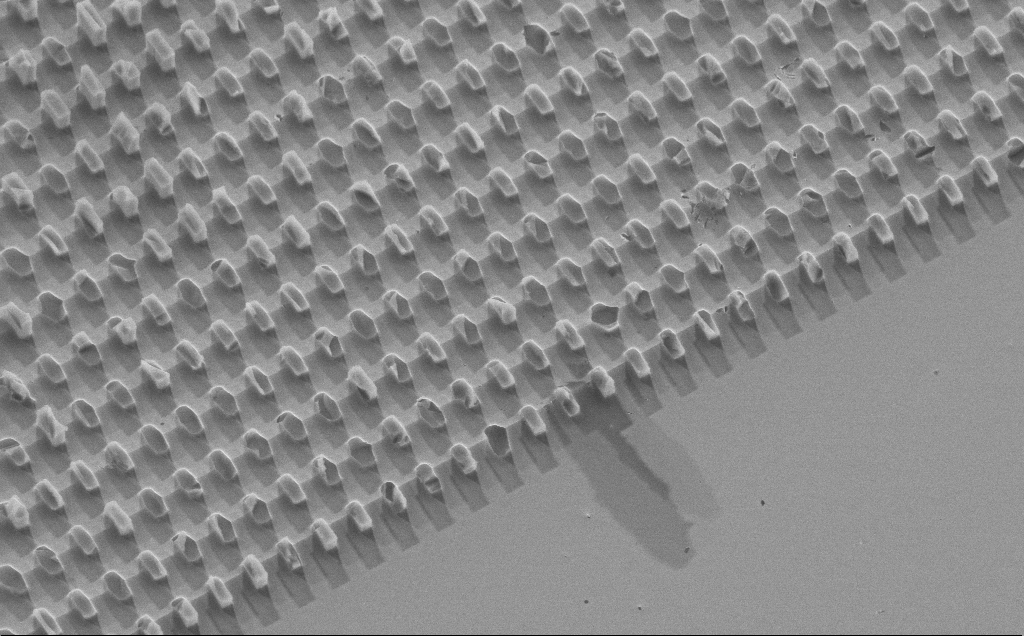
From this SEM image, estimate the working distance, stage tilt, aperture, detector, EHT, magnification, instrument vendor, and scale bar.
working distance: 9 mm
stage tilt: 32.4°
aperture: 30 µm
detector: SE2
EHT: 10 kV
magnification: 3.85 K X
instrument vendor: Zeiss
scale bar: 10000 nm